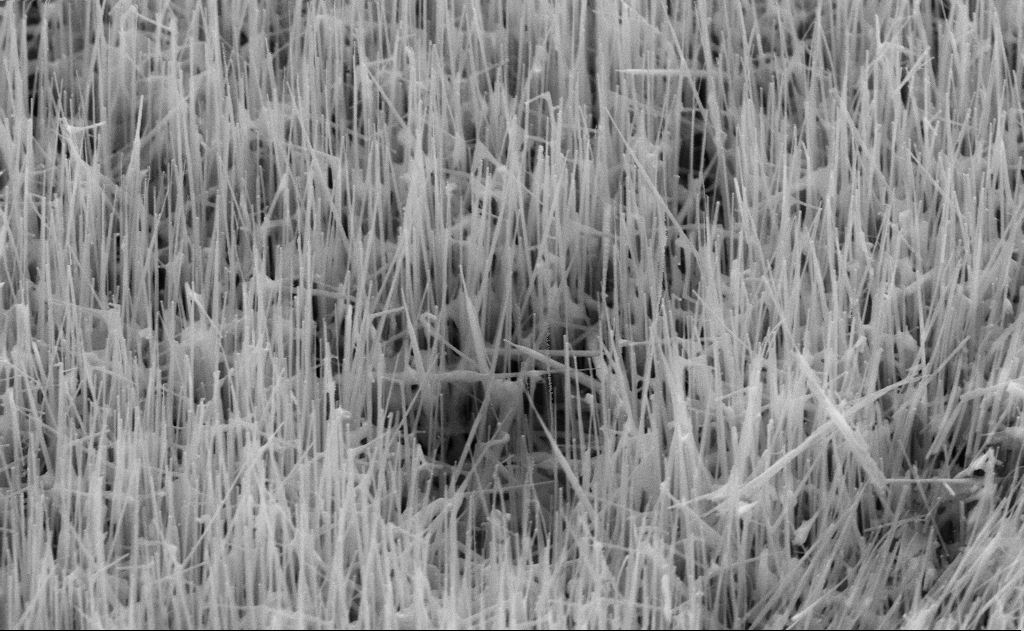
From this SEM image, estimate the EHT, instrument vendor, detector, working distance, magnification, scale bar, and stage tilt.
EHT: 10 kV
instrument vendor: Zeiss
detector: SE2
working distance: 11 mm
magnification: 40 K X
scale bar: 1000 nm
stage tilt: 45°